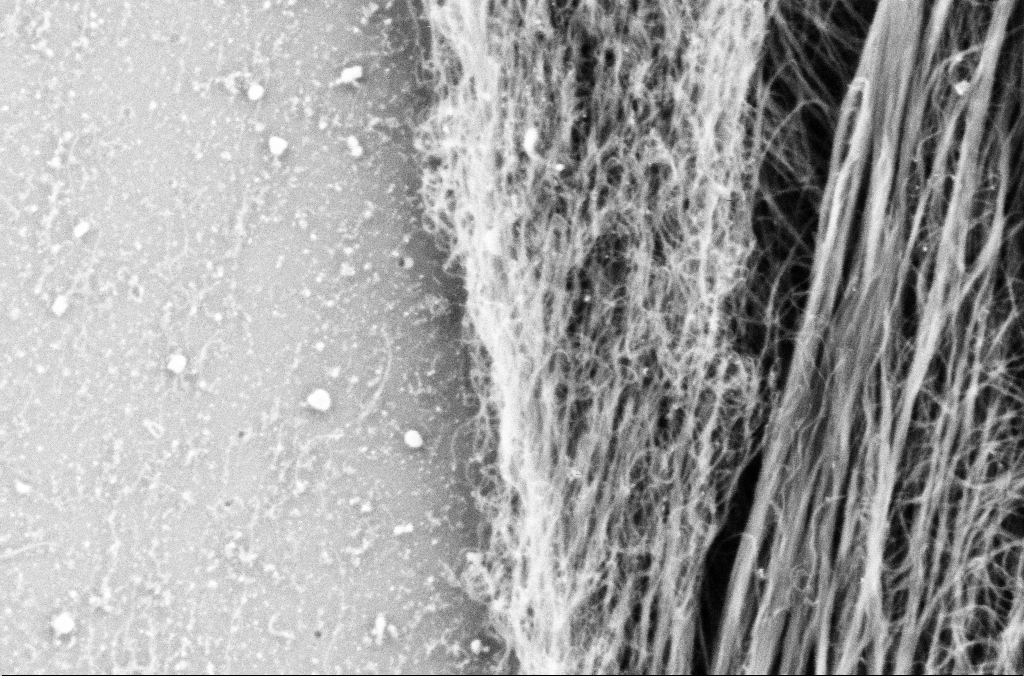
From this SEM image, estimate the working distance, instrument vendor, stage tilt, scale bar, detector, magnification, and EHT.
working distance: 5.7 mm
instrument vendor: Zeiss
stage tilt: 0°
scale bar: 200 nm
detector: SE2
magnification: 100 K X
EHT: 1.8 kV